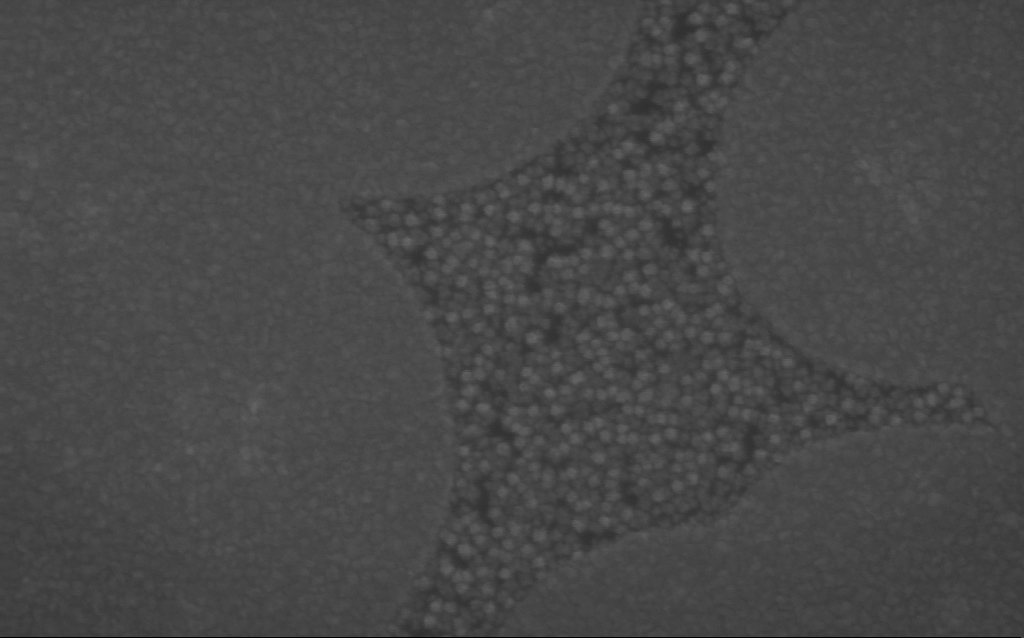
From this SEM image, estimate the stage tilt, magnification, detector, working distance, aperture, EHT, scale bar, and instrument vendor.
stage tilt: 0°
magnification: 316.42 K X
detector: InLens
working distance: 4 mm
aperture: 30 µm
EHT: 7 kV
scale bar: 200 nm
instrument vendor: Zeiss